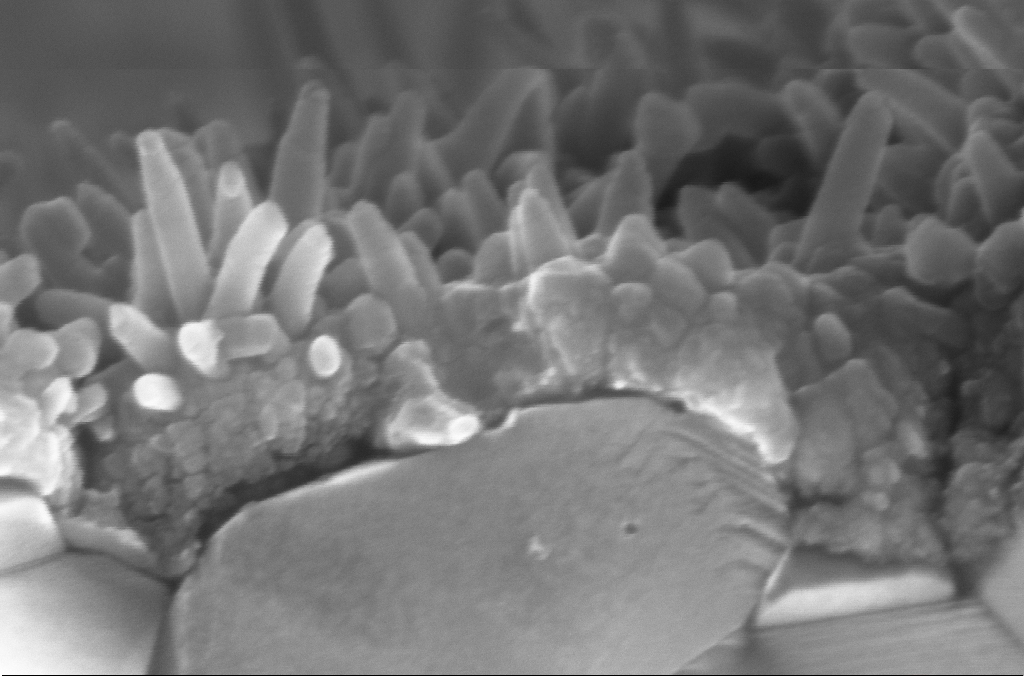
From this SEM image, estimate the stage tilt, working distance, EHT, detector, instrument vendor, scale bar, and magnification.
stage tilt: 4.1°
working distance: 8.4 mm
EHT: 10 kV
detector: InLens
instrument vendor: Zeiss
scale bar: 200 nm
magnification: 281.86 K X